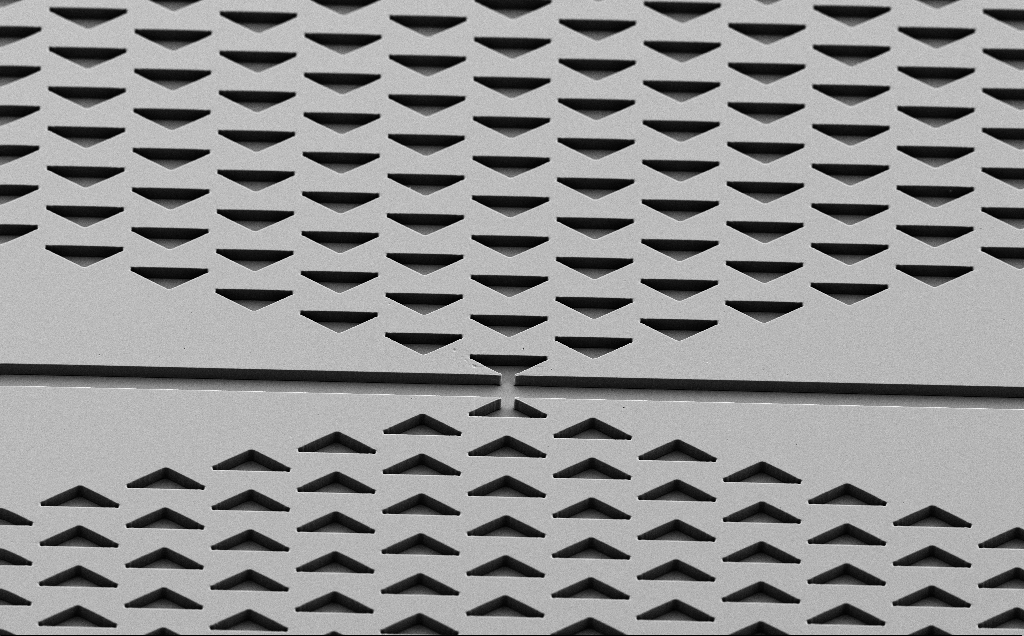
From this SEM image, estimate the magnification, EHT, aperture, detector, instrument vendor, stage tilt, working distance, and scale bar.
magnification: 0.803 K X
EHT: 5 kV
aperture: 30 µm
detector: SE2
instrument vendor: Zeiss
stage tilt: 40°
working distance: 9 mm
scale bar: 20000 nm